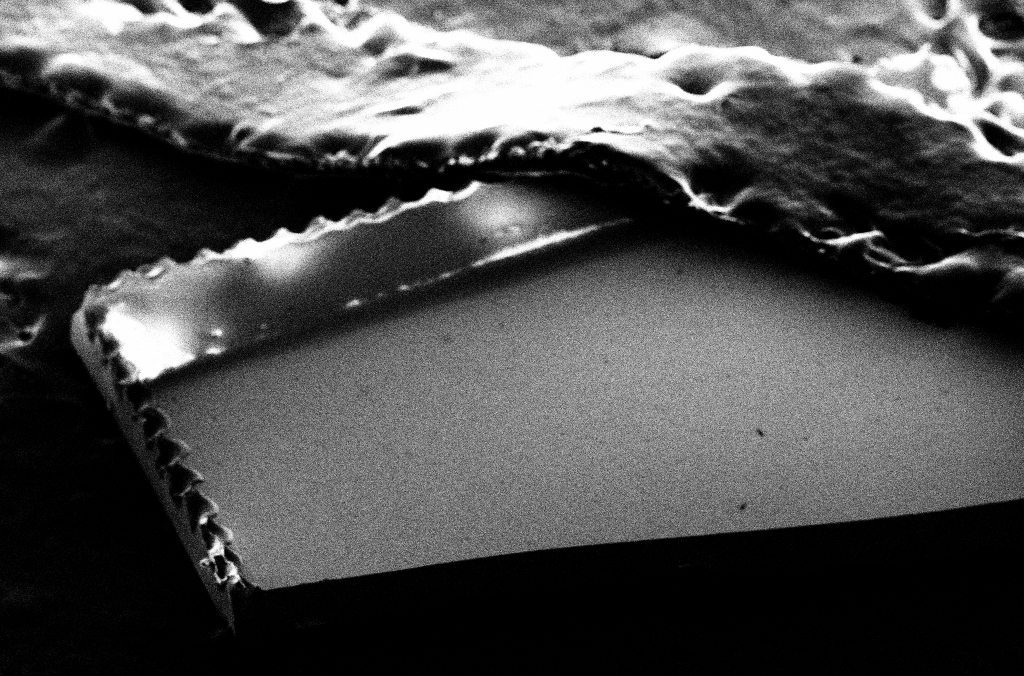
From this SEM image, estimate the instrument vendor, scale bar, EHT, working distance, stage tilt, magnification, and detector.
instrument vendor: Zeiss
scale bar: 100000 nm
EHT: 10 kV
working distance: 6.6 mm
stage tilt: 45°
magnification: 0.125 K X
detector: SE2